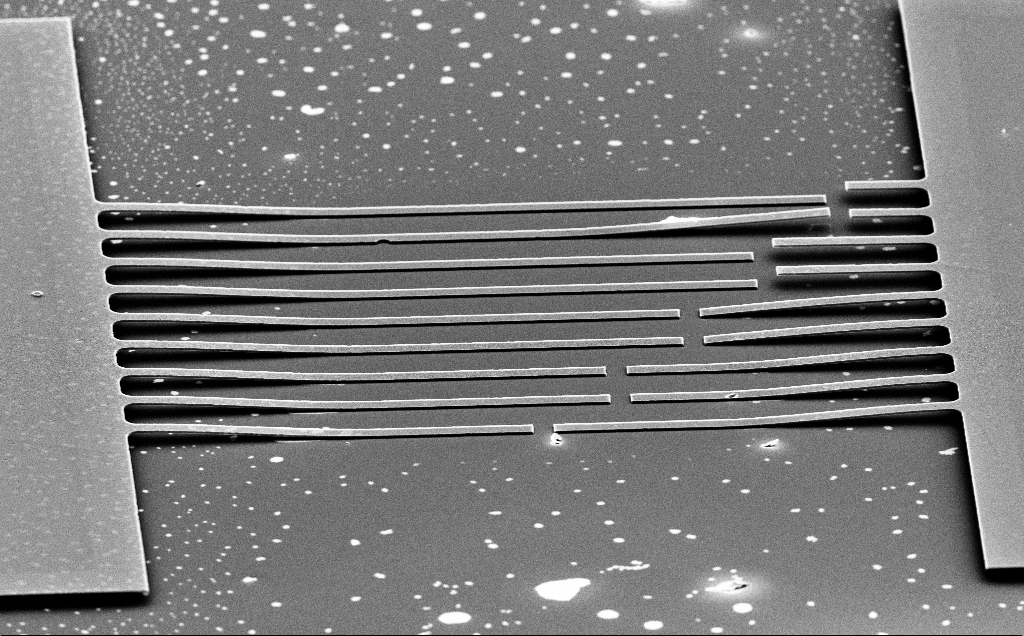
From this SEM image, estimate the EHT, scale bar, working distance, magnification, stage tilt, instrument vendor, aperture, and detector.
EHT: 10 kV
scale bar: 20000 nm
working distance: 18 mm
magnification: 1.19 K X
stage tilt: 65.4°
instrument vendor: Zeiss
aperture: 30 µm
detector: SE2